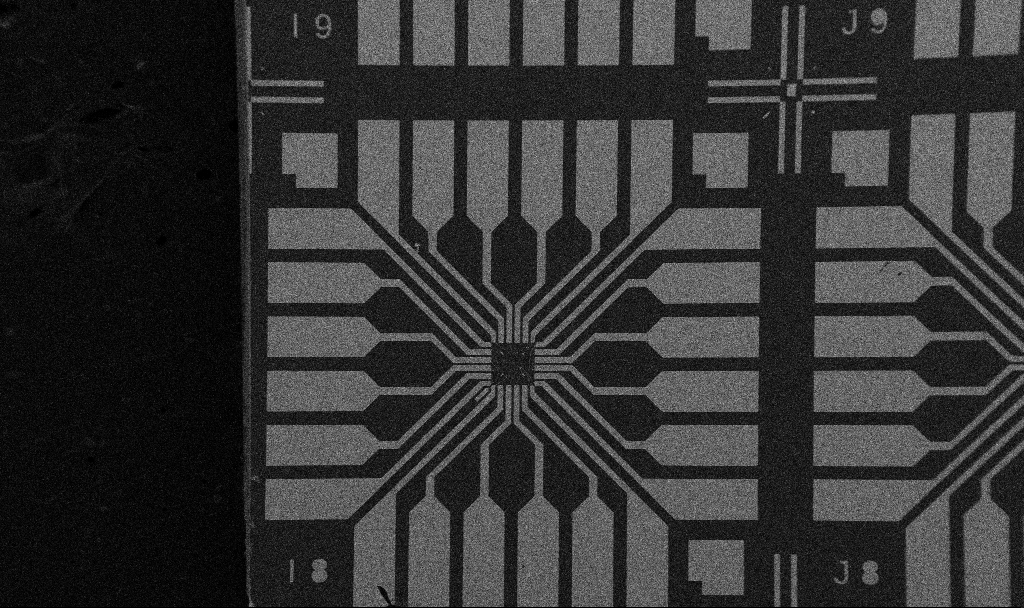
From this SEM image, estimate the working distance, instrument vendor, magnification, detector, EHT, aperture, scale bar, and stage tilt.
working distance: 10.7 mm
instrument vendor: Zeiss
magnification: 0.1 K X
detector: SE2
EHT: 5 kV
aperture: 30 µm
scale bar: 200000 nm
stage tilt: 0°